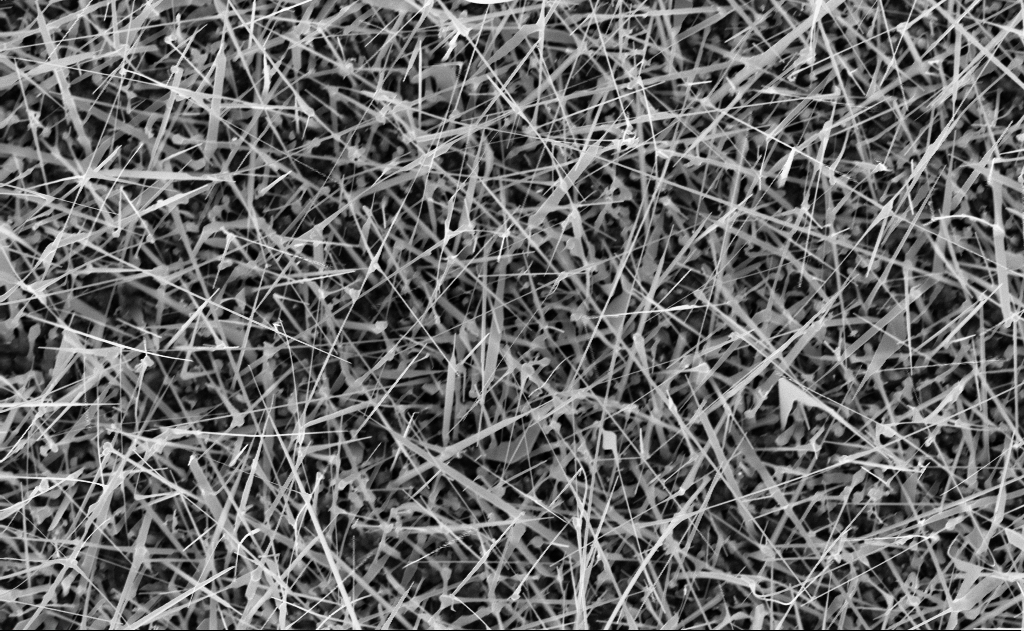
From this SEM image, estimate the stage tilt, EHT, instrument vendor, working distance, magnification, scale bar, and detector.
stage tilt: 0°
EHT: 10 kV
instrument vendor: Zeiss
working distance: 10 mm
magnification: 20 K X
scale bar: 2000 nm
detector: InLens